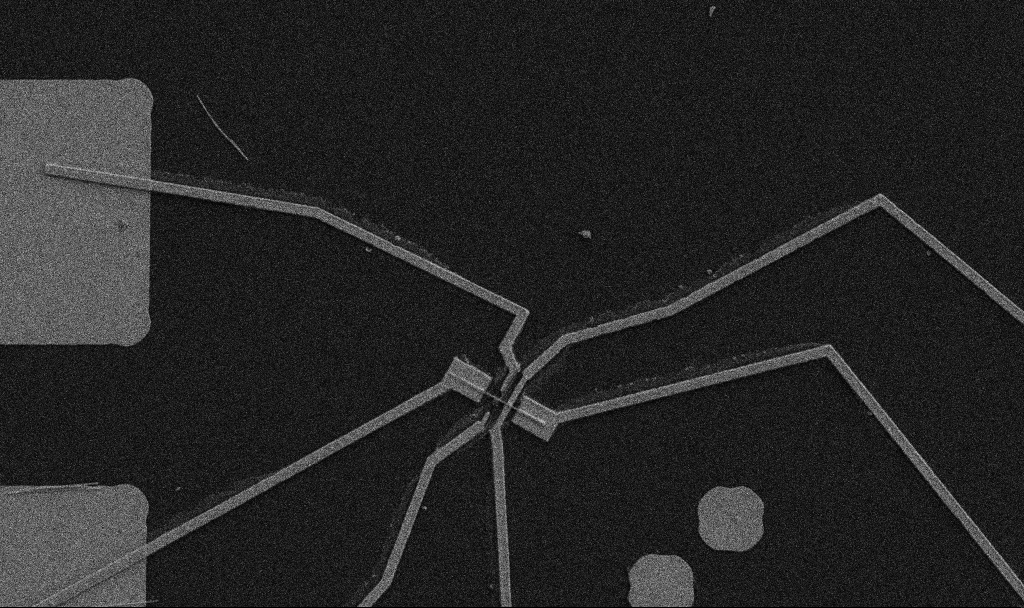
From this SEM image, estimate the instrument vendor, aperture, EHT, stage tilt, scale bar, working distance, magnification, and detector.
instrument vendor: Zeiss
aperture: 30 µm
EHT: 5 kV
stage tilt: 0°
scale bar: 10000 nm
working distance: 10.7 mm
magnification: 5 K X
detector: SE2